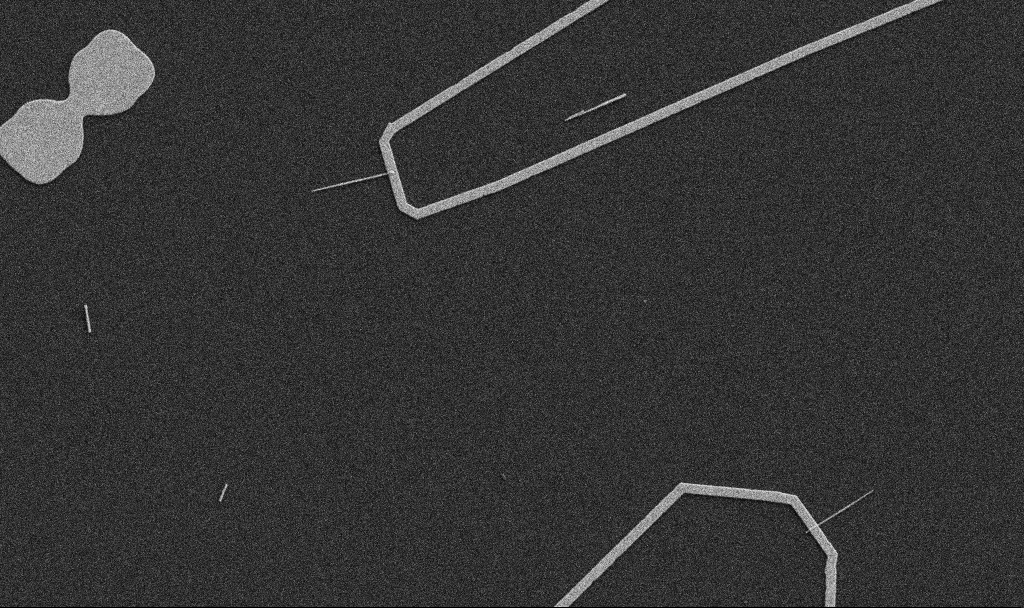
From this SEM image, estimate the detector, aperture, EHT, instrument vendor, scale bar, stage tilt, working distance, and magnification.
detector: SE2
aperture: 30 µm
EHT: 5 kV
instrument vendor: Zeiss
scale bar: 10000 nm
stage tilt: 0°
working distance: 10.7 mm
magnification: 5 K X